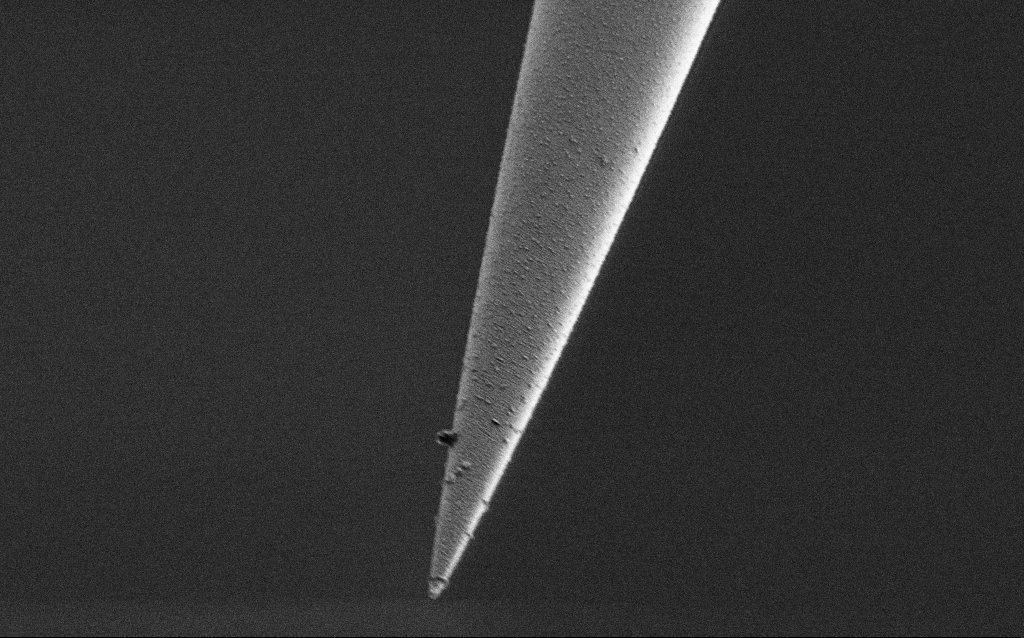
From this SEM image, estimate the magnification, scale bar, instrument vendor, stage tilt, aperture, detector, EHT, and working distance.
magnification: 25 K X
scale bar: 2000 nm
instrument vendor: Zeiss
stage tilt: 45°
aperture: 30 µm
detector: SE2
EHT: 1 kV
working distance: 6.5 mm